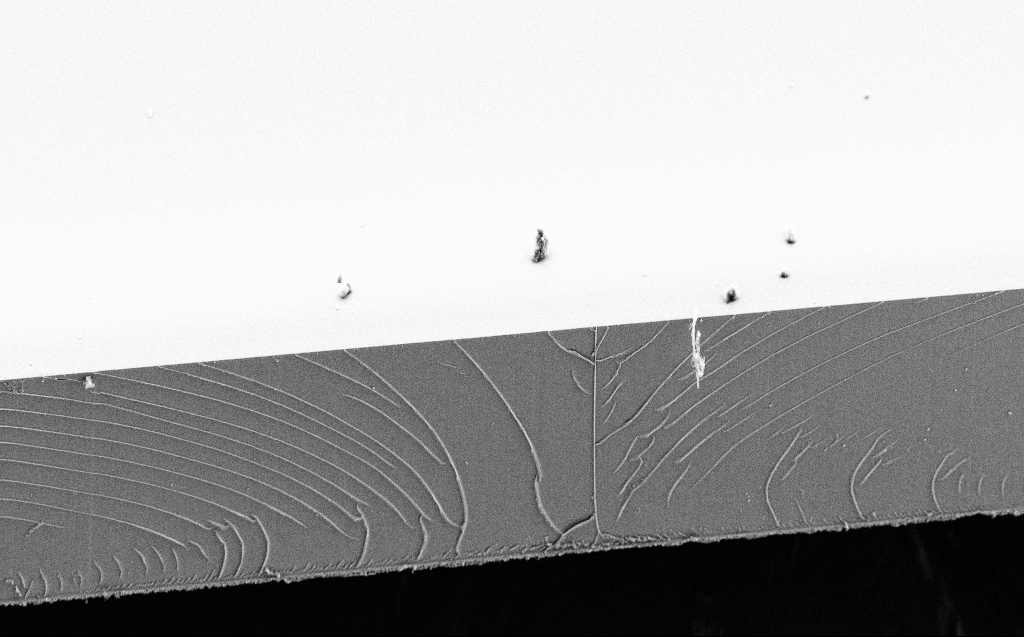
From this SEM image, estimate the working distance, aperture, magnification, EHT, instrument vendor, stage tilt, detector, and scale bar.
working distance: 5 mm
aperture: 30 µm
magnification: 1.06 K X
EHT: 10 kV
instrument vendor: Zeiss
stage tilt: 45°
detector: SE2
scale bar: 20000 nm